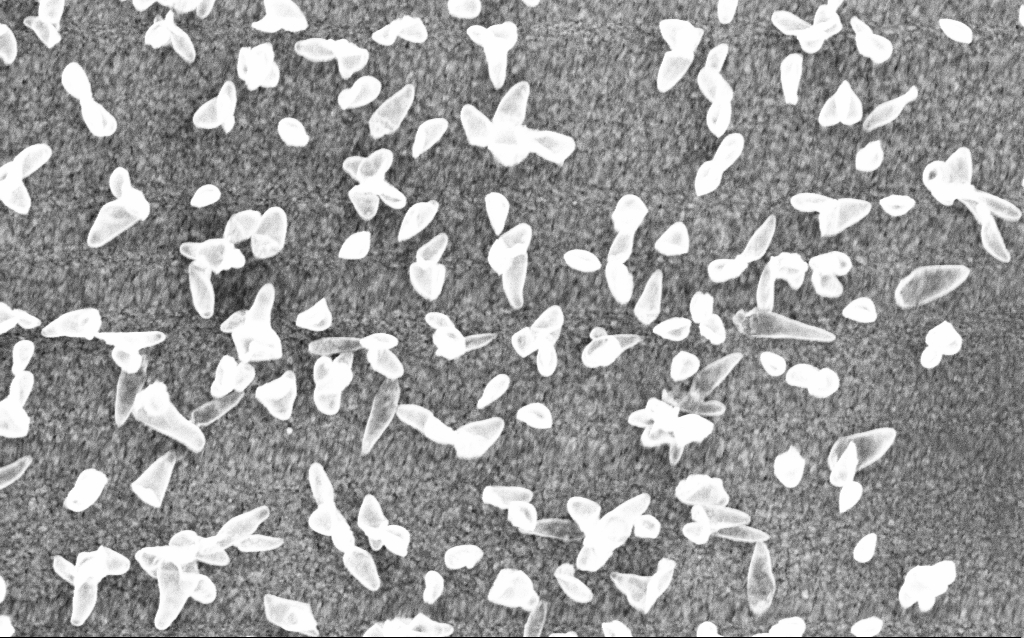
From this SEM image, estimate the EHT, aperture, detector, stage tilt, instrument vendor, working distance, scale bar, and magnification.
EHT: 5 kV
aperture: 30 µm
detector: InLens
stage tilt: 0°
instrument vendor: Zeiss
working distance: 6 mm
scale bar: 200 nm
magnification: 77.69 K X